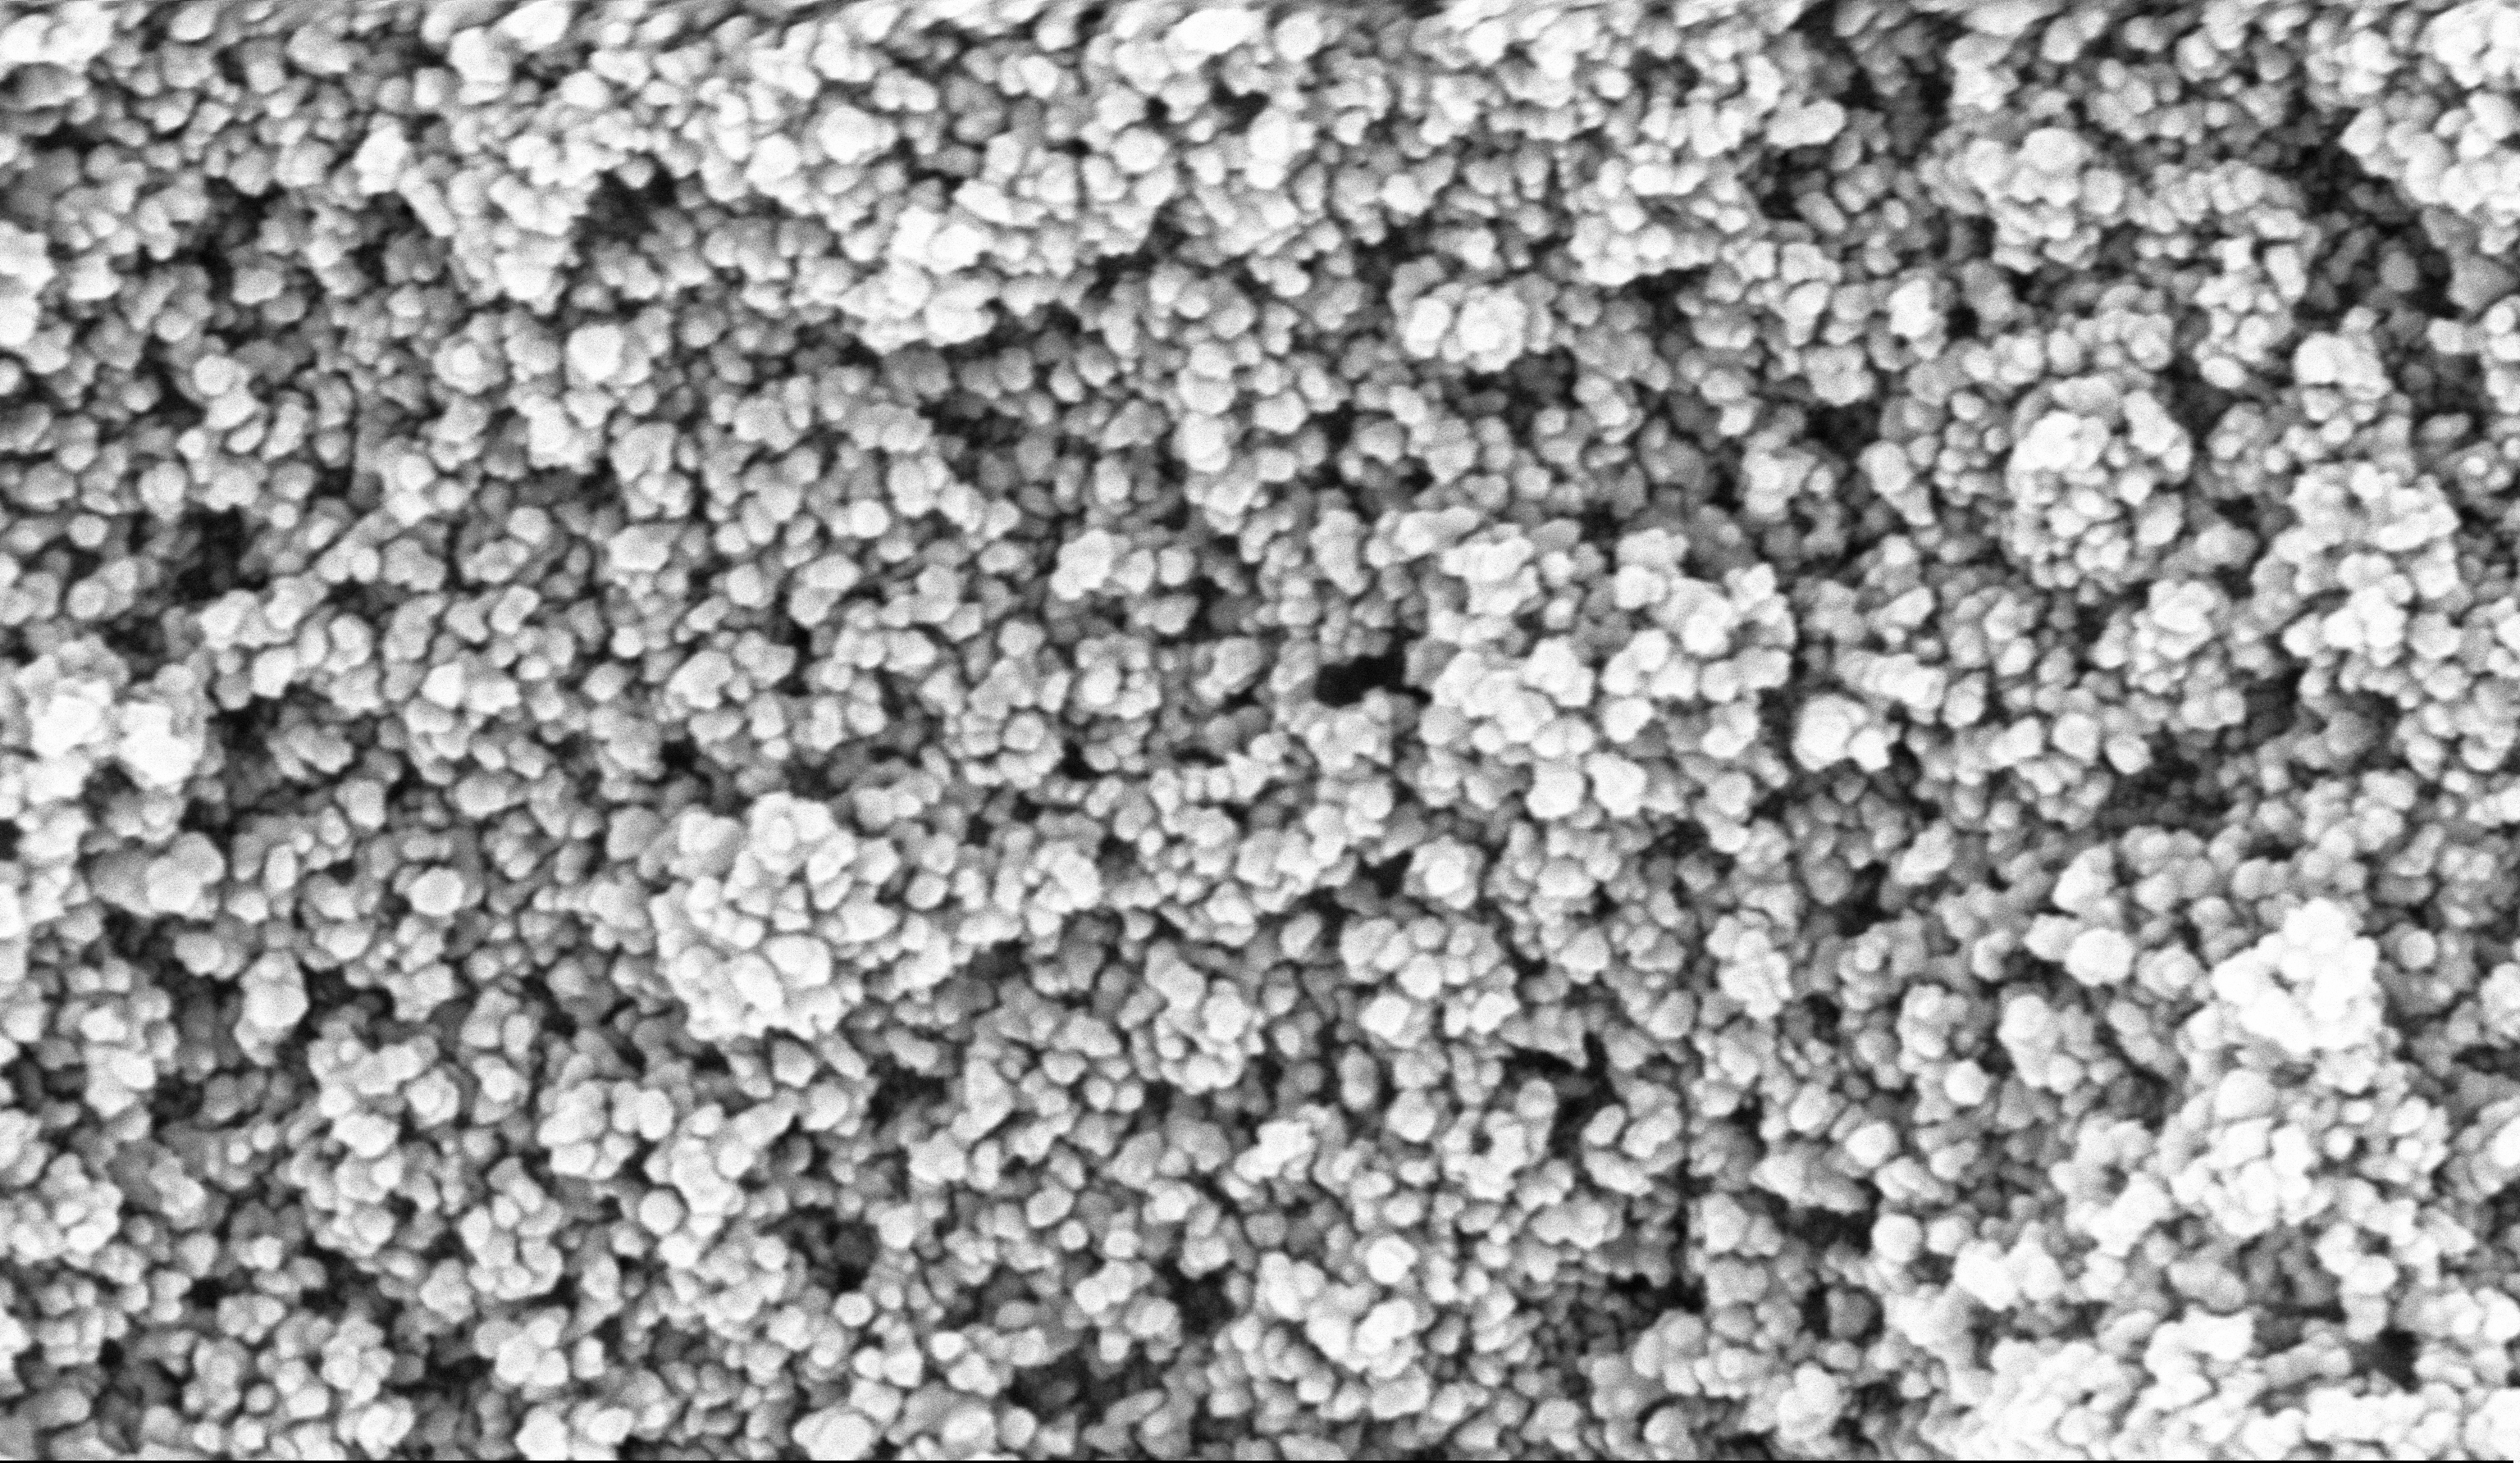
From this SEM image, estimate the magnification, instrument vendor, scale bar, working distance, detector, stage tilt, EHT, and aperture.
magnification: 135 K X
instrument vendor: Zeiss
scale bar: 100 nm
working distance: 5.1 mm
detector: InLens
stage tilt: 0°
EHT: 10 kV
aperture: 30 µm